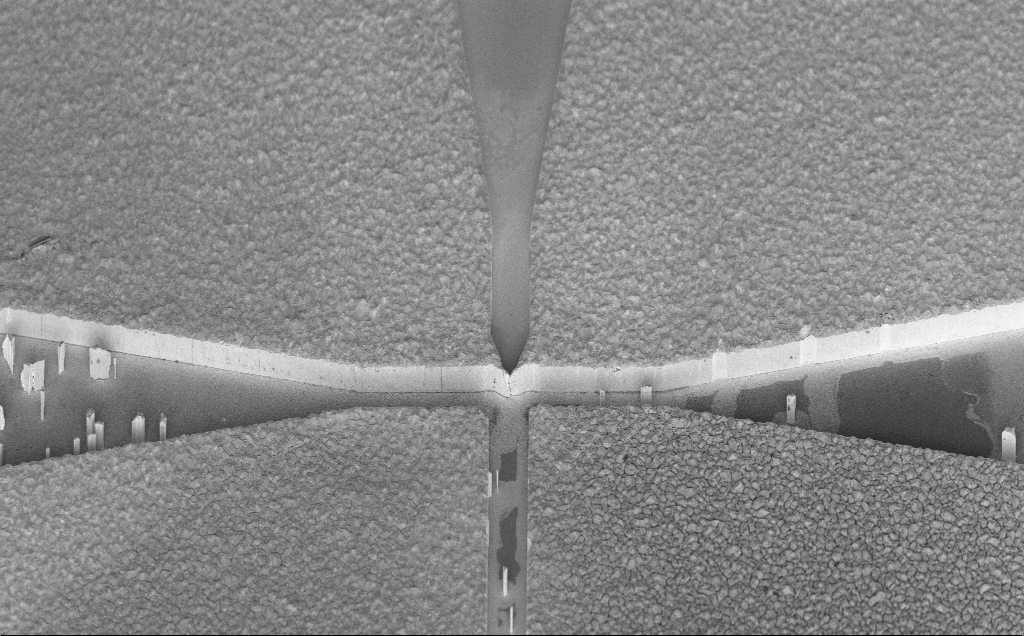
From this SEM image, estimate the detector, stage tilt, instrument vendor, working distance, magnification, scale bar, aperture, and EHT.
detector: InLens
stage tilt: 45°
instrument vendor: Zeiss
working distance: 9 mm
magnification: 1.44 K X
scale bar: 10000 nm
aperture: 30 µm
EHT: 5 kV